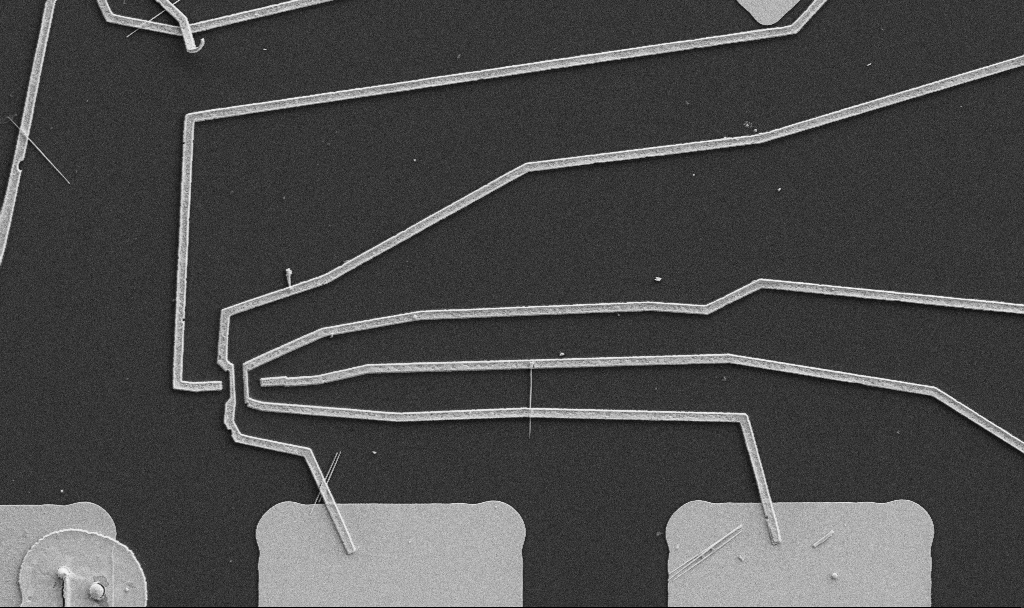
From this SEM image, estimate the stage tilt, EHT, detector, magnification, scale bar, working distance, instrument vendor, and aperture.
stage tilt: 0°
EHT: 5 kV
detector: SE2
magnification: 5 K X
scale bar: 10000 nm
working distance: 10.7 mm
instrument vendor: Zeiss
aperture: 30 µm